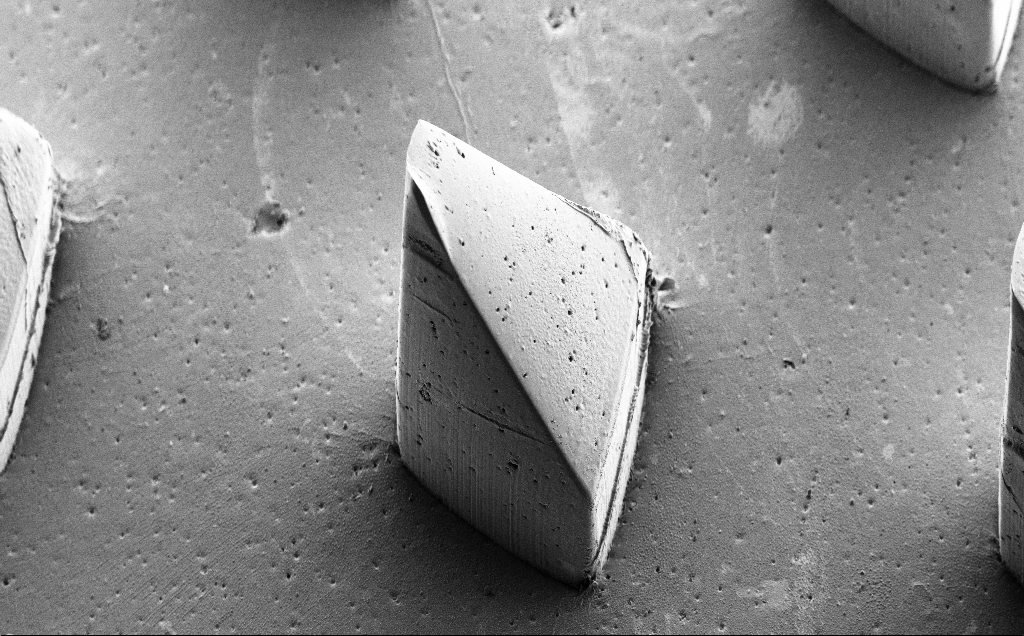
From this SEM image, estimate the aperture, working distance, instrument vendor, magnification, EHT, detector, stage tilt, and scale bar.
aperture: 30 µm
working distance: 8 mm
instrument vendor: Zeiss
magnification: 0.241 K X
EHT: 5 kV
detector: SE2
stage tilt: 39°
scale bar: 200000 nm